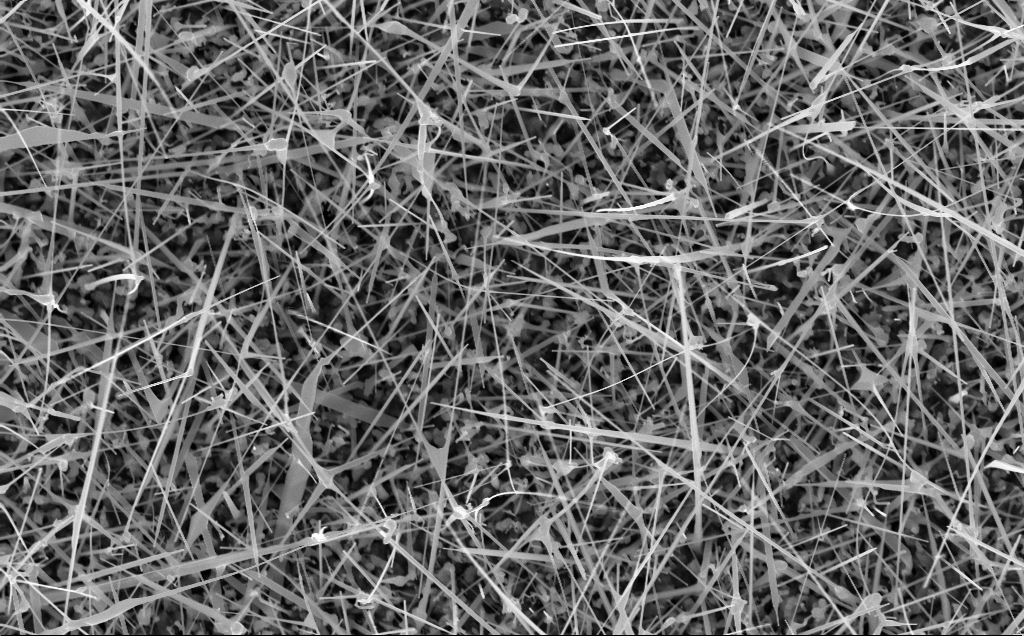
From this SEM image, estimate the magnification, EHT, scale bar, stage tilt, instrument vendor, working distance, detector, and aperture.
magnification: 20 K X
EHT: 10 kV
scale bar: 2000 nm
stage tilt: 0°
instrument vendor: Zeiss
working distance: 8 mm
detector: InLens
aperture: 30 µm